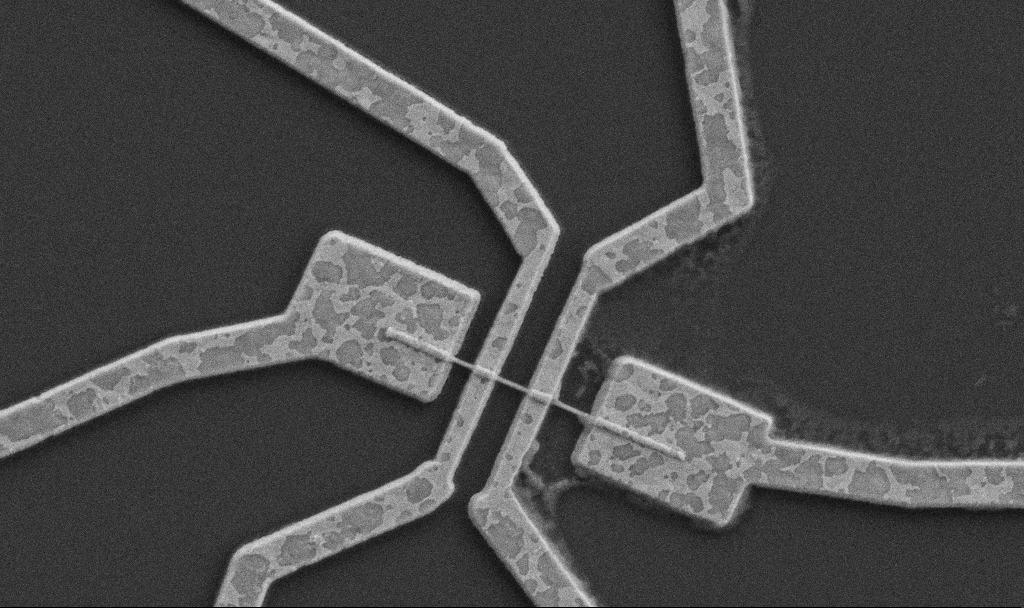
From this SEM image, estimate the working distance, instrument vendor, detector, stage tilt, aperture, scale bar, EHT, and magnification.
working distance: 8.7 mm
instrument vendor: Zeiss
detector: SE2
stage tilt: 0°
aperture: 30 µm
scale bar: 1000 nm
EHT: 5 kV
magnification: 20 K X